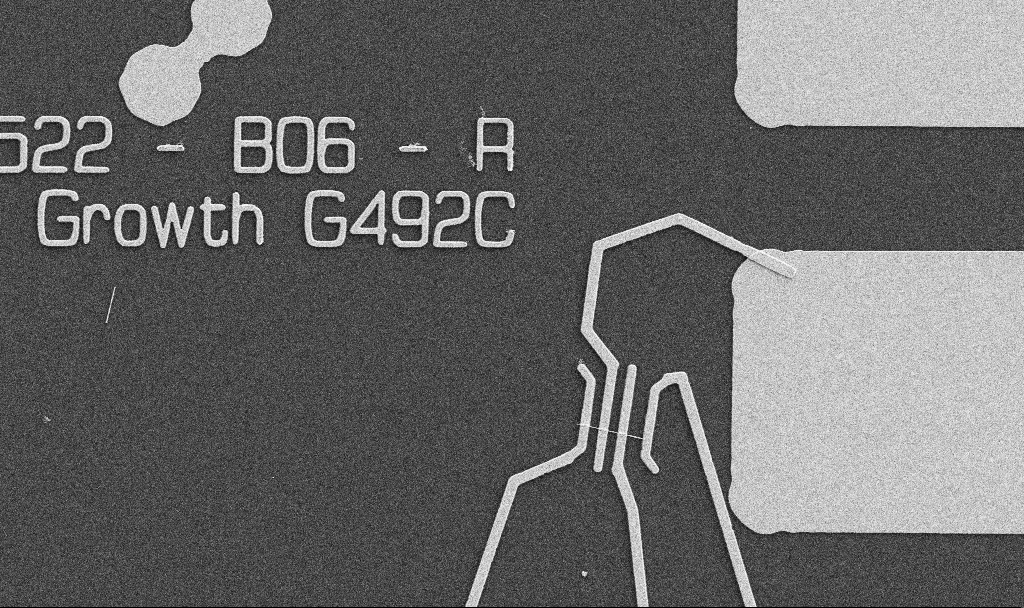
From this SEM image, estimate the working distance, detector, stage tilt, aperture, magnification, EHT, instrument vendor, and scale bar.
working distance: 10.7 mm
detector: SE2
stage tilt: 0°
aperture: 30 µm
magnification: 5 K X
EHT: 5 kV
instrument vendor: Zeiss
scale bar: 10000 nm